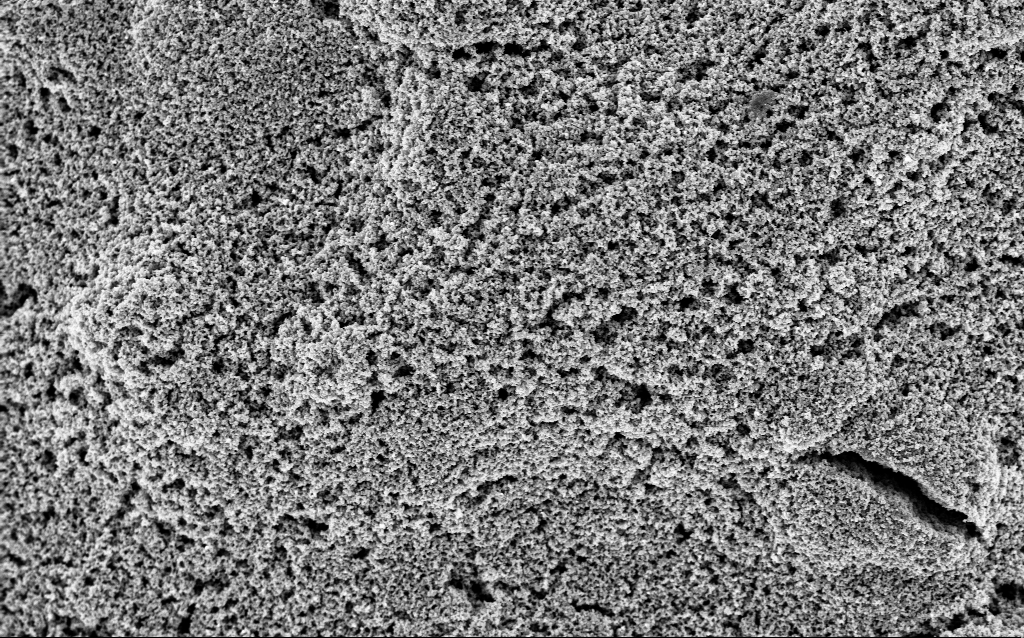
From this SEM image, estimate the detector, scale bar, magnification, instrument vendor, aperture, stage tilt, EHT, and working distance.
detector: InLens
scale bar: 1000 nm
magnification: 23.9 K X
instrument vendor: Zeiss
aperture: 30 µm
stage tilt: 0°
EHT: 3 kV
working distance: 7.5 mm